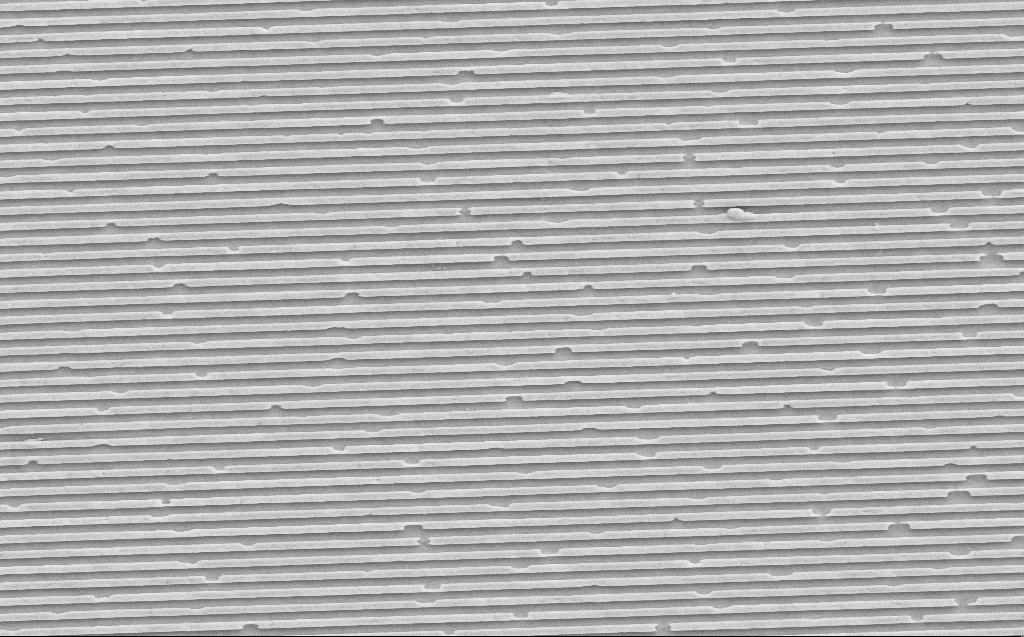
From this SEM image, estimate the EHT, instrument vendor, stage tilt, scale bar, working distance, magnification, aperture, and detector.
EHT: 10 kV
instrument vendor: Zeiss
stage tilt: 0°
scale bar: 2000 nm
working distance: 10 mm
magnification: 11.58 K X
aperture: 30 µm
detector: SE2